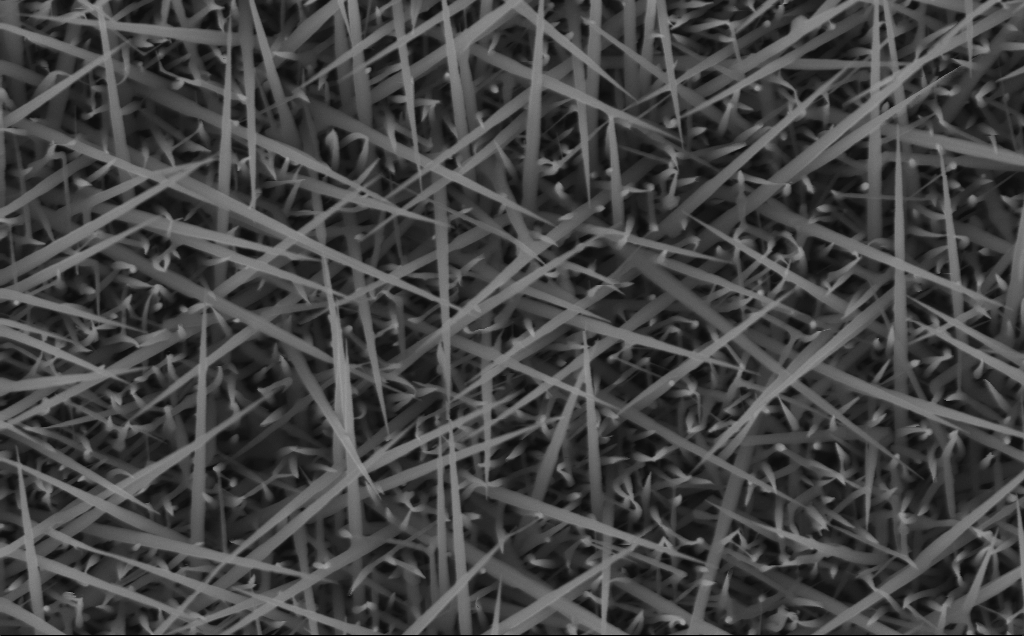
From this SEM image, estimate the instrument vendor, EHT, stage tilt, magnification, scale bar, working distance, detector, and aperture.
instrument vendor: Zeiss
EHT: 10 kV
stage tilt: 0°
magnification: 80 K X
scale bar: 200 nm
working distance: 6 mm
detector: InLens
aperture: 30 µm